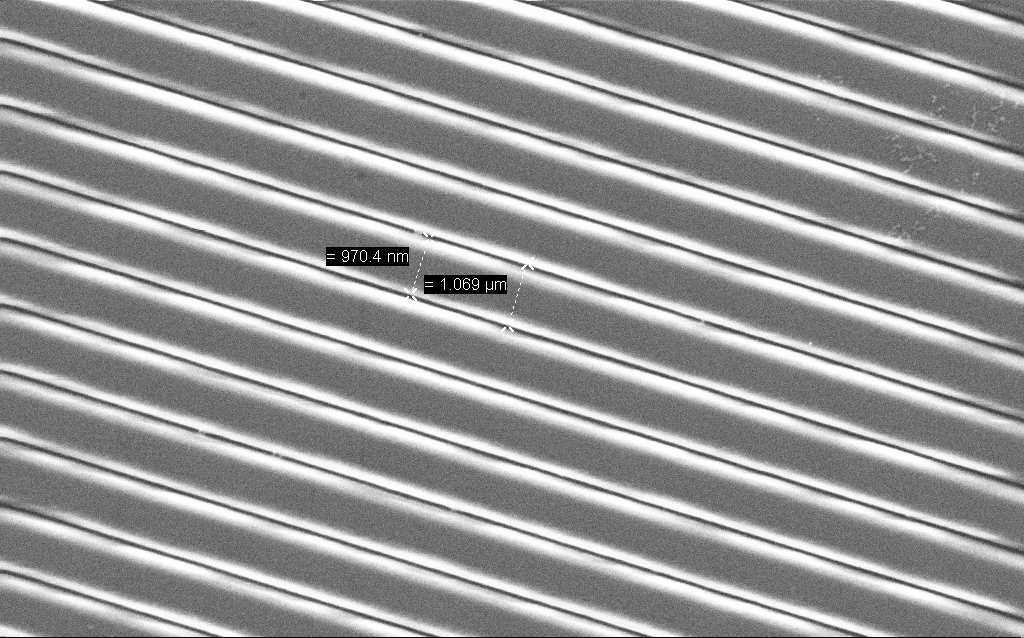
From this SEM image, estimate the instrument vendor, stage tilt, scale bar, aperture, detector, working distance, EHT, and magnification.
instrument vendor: Zeiss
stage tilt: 0°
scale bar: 2000 nm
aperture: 30 µm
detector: SE2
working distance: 6.6 mm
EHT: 1.5 kV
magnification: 22.38 K X